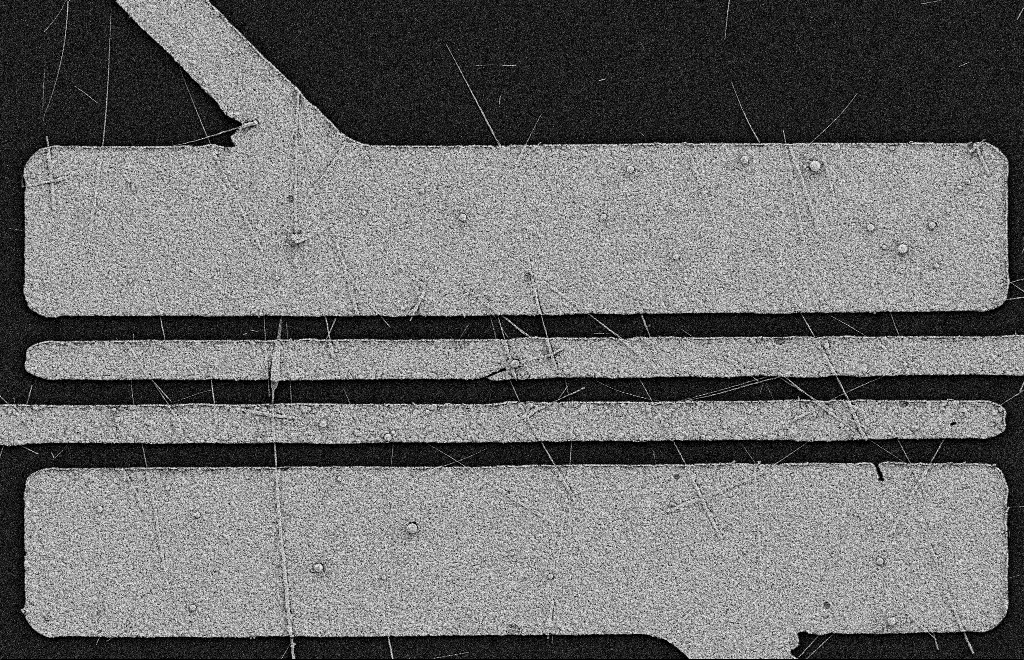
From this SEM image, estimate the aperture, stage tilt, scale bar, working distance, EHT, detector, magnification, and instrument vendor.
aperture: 20 µm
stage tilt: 0°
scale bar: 2000 nm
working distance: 11 mm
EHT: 2 kV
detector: SE2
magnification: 5.86 K X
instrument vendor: Zeiss